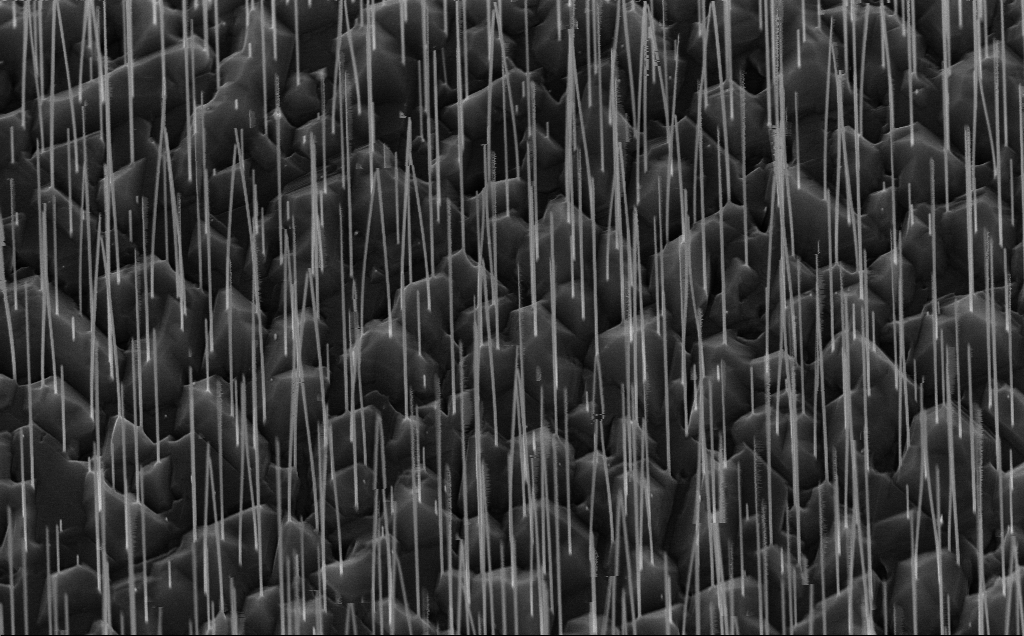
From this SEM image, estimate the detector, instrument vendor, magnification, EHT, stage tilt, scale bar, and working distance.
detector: InLens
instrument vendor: Zeiss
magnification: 32.02 K X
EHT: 10 kV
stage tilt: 44.9°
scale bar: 2000 nm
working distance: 7 mm